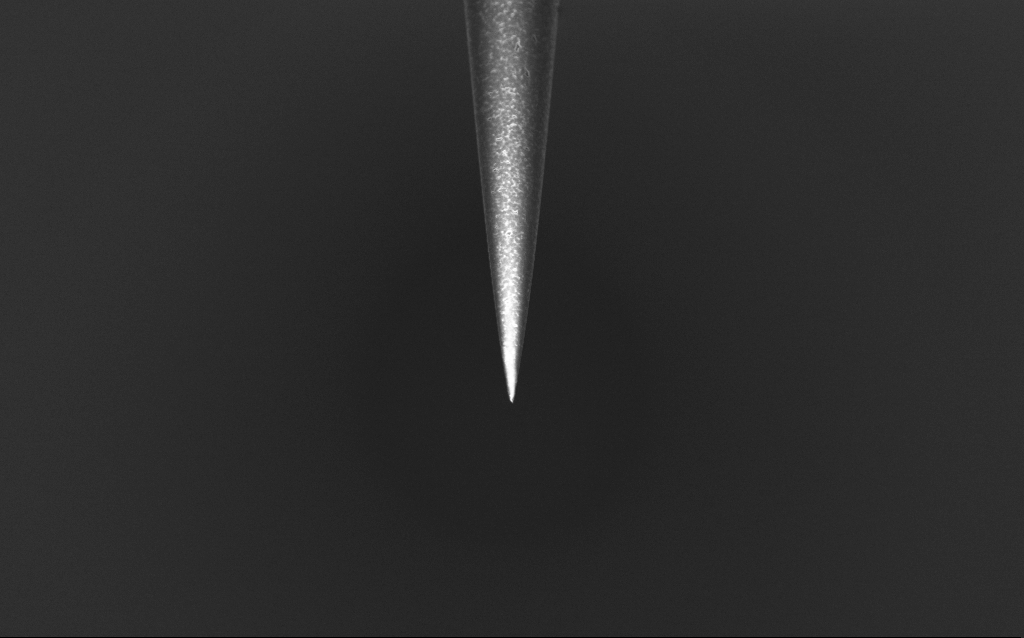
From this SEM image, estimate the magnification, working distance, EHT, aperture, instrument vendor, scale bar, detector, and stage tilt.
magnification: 10 K X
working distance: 6 mm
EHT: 1 kV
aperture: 30 µm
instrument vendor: Zeiss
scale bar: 2000 nm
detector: InLens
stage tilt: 45°